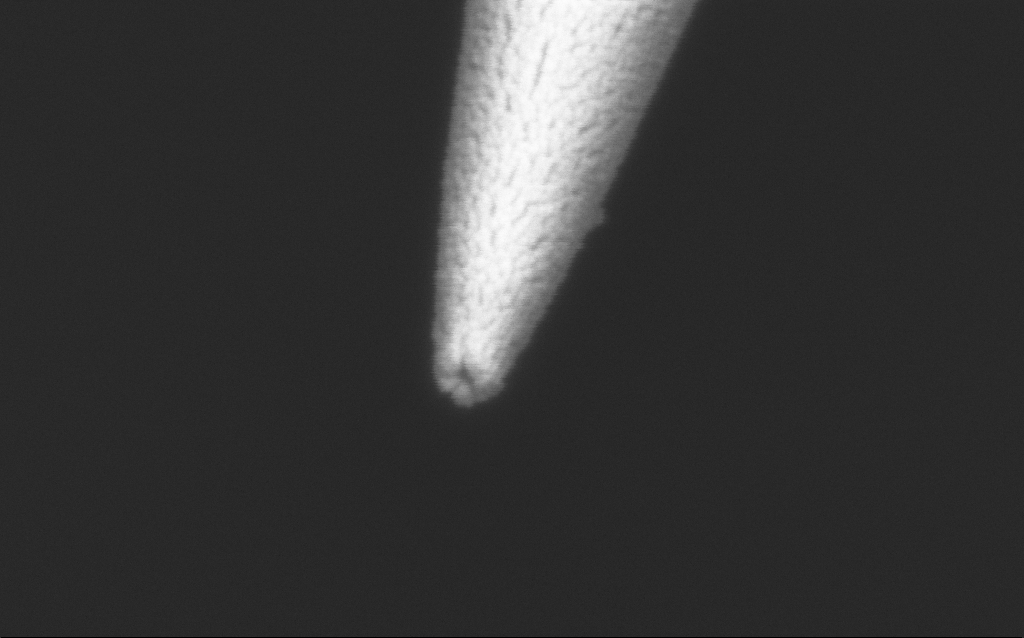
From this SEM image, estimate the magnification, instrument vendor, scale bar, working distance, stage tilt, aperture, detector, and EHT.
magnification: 250 K X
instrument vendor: Zeiss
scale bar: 100 nm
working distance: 7.6 mm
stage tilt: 45°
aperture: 30 µm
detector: InLens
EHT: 2 kV